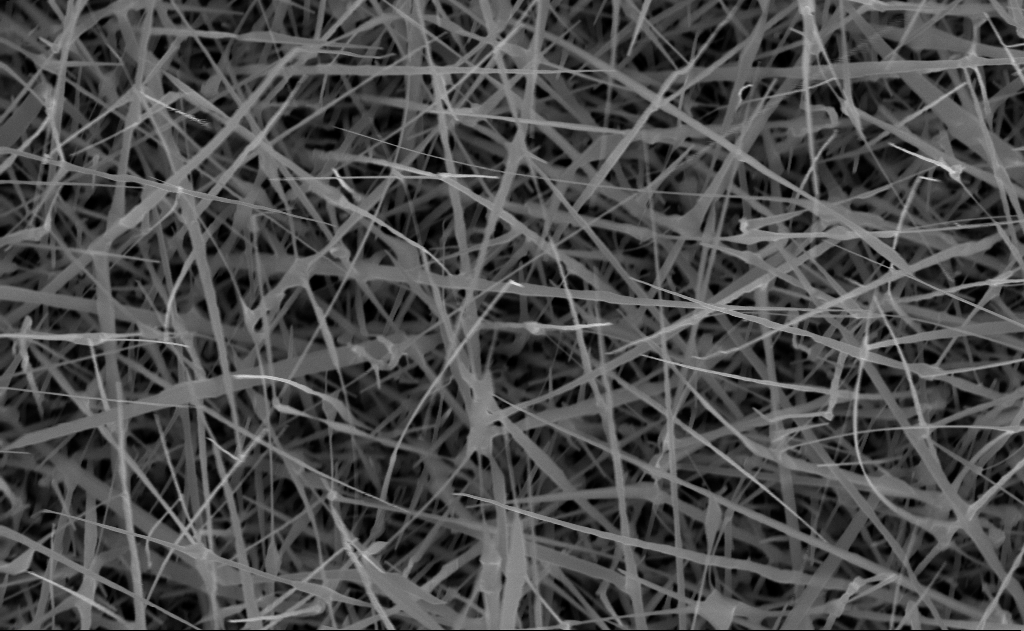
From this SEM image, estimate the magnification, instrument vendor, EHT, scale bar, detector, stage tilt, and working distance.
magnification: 40 K X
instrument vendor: Zeiss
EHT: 10 kV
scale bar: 1000 nm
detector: InLens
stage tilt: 0°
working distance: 10 mm